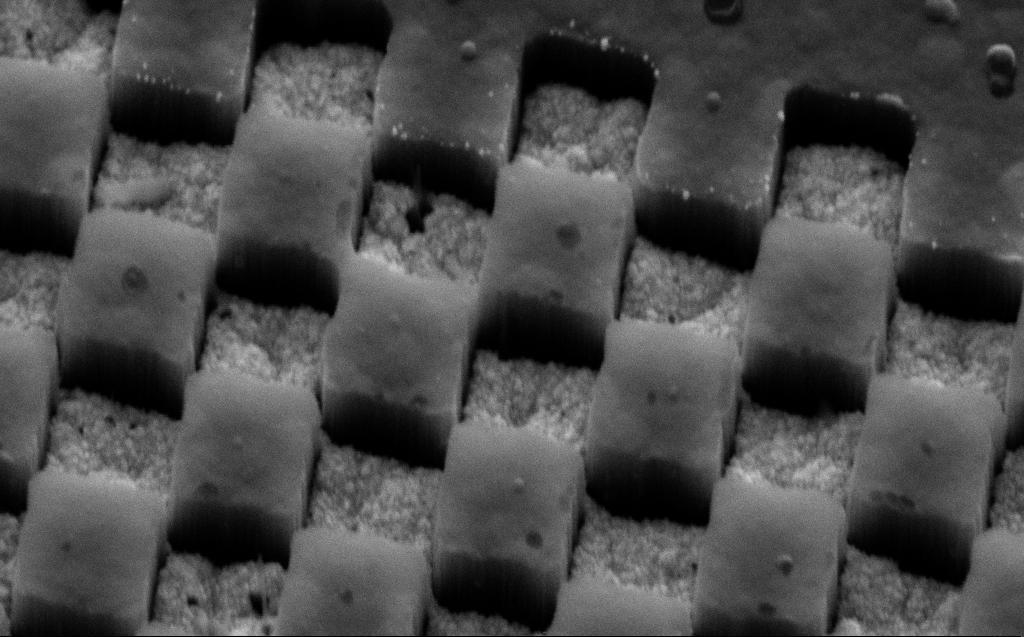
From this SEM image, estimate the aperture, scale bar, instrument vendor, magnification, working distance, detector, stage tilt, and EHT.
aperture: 30 µm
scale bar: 200 nm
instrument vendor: Zeiss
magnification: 98.29 K X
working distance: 9 mm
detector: SE2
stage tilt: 45°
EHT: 5 kV